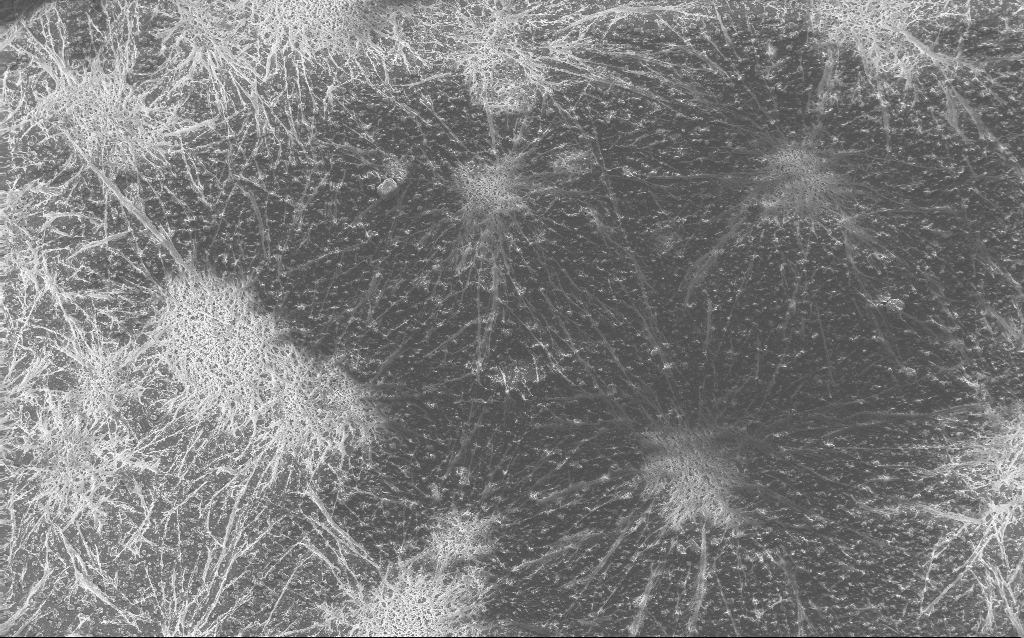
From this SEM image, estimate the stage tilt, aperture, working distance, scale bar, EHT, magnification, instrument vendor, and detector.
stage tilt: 0°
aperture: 30 µm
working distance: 2.8 mm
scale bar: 100000 nm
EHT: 10 kV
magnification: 0.235 K X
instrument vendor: Zeiss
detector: InLens